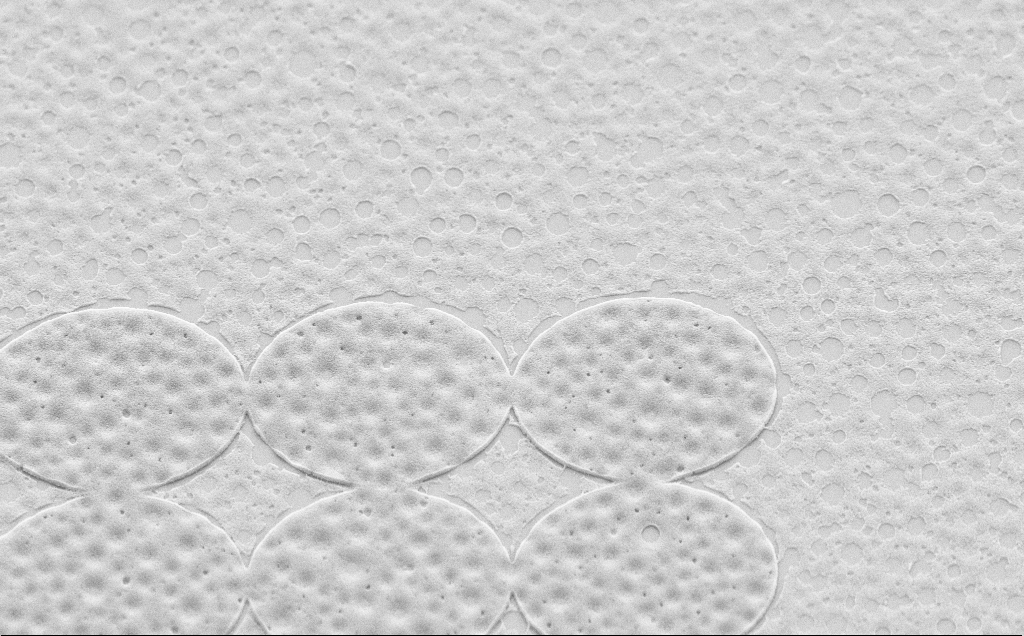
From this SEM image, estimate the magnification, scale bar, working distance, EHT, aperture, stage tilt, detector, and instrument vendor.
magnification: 9.81 K X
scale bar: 2000 nm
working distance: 6 mm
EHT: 5 kV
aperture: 30 µm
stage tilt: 45°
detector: SE2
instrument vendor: Zeiss